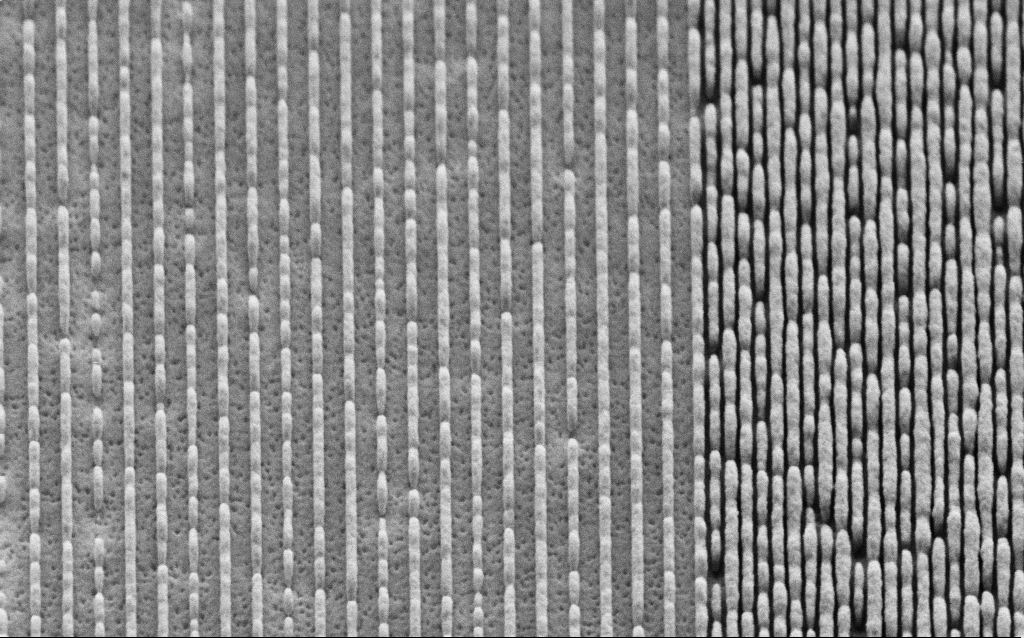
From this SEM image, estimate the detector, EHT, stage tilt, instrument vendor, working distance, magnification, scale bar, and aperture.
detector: SE2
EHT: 3 kV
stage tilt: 45°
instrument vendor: Zeiss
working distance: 6.6 mm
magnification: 58.09 K X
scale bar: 1000 nm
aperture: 30 µm